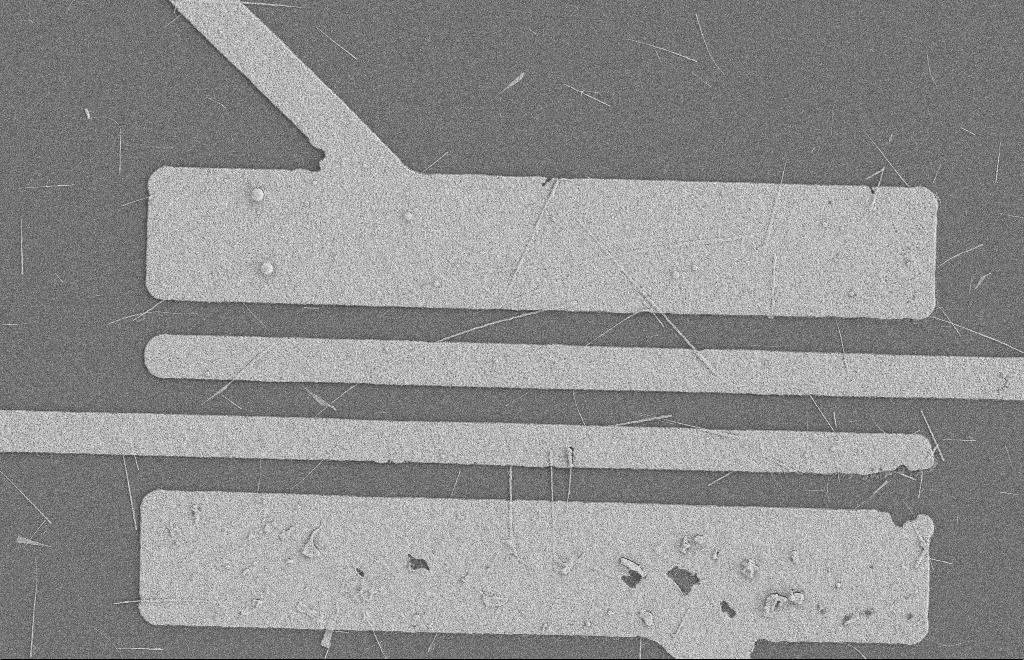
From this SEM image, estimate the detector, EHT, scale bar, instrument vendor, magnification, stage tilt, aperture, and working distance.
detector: SE2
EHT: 2 kV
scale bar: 2000 nm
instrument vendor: Zeiss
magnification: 4.77 K X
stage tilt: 0°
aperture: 20 µm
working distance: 8 mm